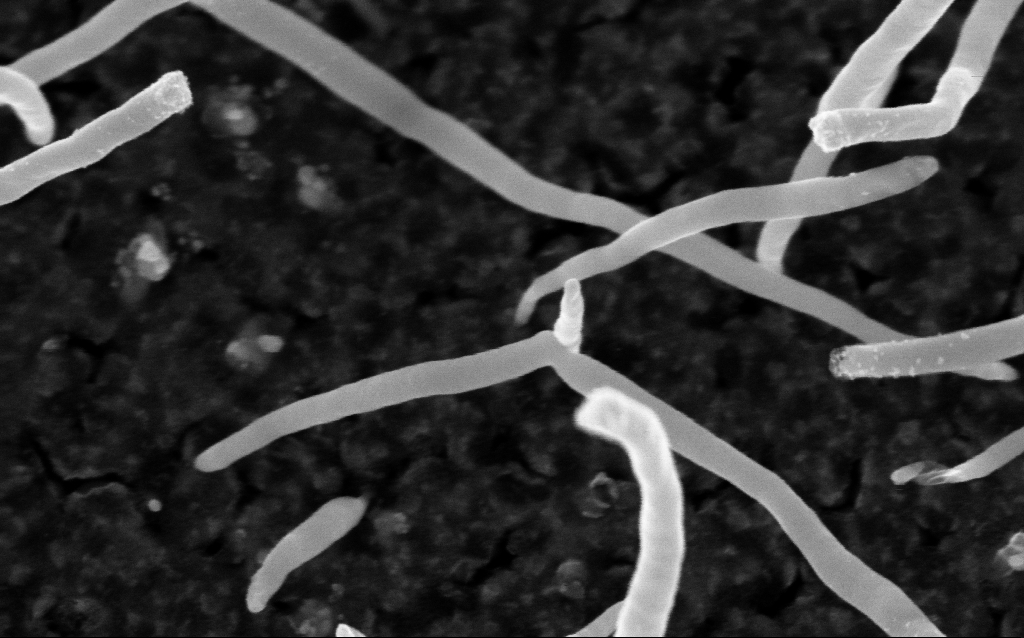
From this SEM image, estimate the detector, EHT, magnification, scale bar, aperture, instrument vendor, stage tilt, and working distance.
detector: InLens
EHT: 5 kV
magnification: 200 K X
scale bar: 100 nm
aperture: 30 µm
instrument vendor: Zeiss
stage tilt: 0°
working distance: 1.7 mm